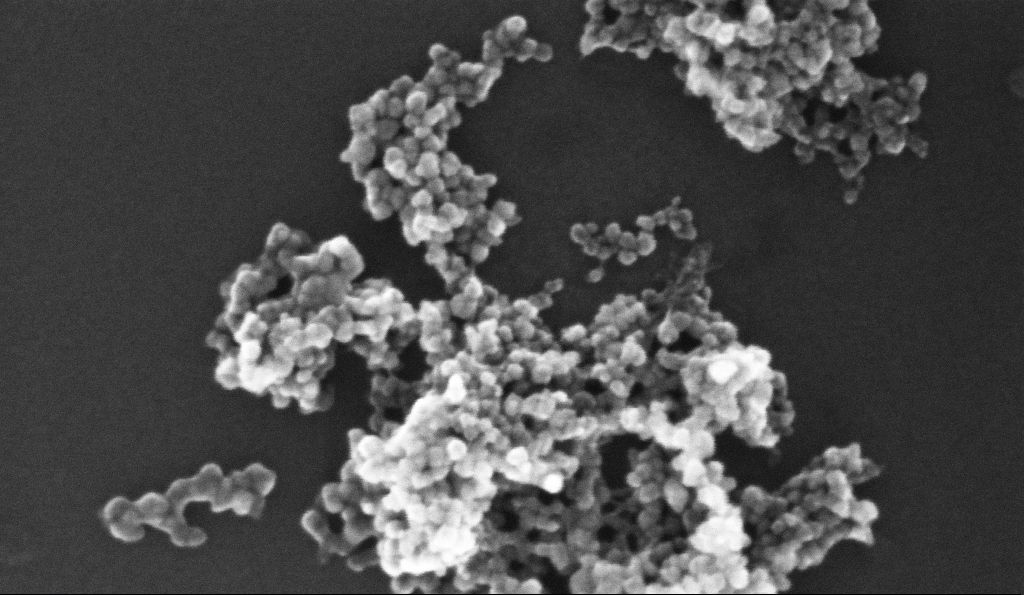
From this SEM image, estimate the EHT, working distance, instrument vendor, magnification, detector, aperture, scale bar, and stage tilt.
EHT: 10 kV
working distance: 5.2 mm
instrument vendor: Zeiss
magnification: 336.79 K X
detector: InLens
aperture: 30 µm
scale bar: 100 nm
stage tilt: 0°